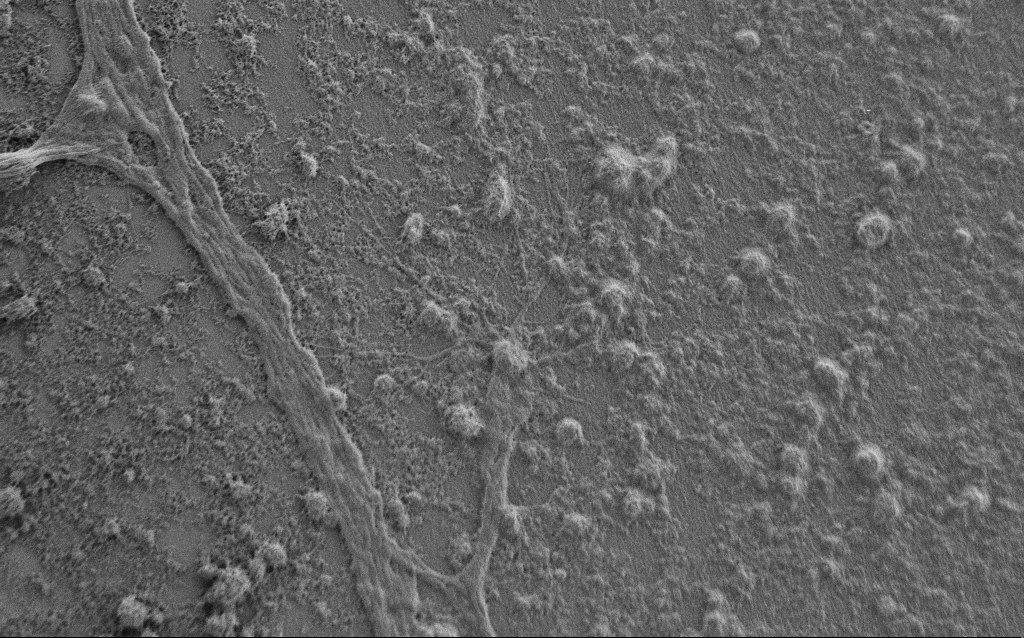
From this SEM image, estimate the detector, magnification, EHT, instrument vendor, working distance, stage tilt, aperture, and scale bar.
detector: SE2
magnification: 5 K X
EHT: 0.9 kV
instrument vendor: Zeiss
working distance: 7 mm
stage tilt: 0°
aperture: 30 µm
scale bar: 10000 nm